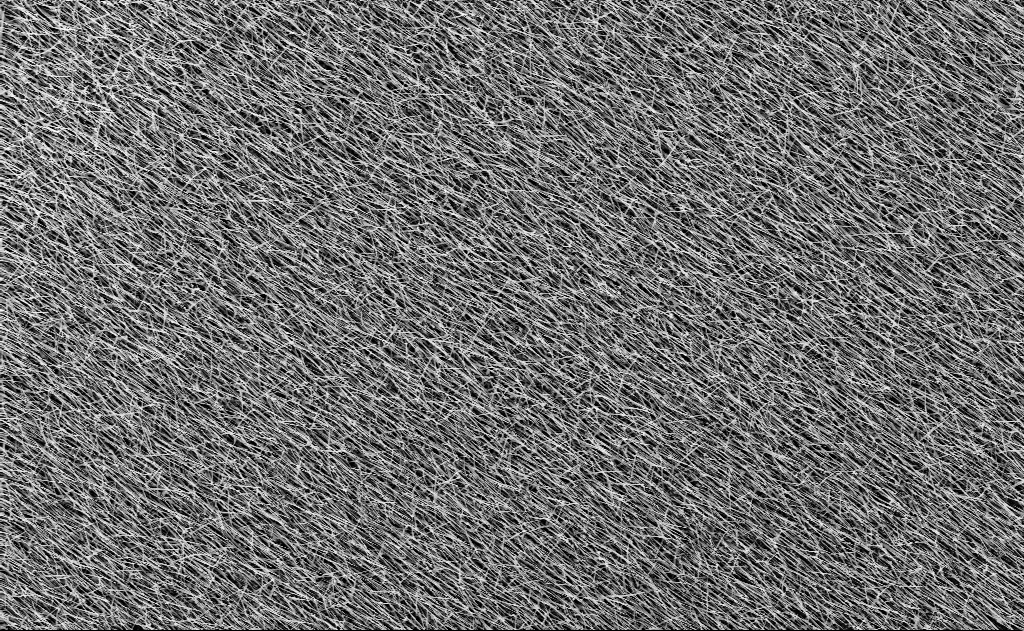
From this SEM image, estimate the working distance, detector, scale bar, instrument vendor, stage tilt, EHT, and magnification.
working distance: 13 mm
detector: InLens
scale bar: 10000 nm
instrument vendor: Zeiss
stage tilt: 0°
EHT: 10 kV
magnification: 5 K X